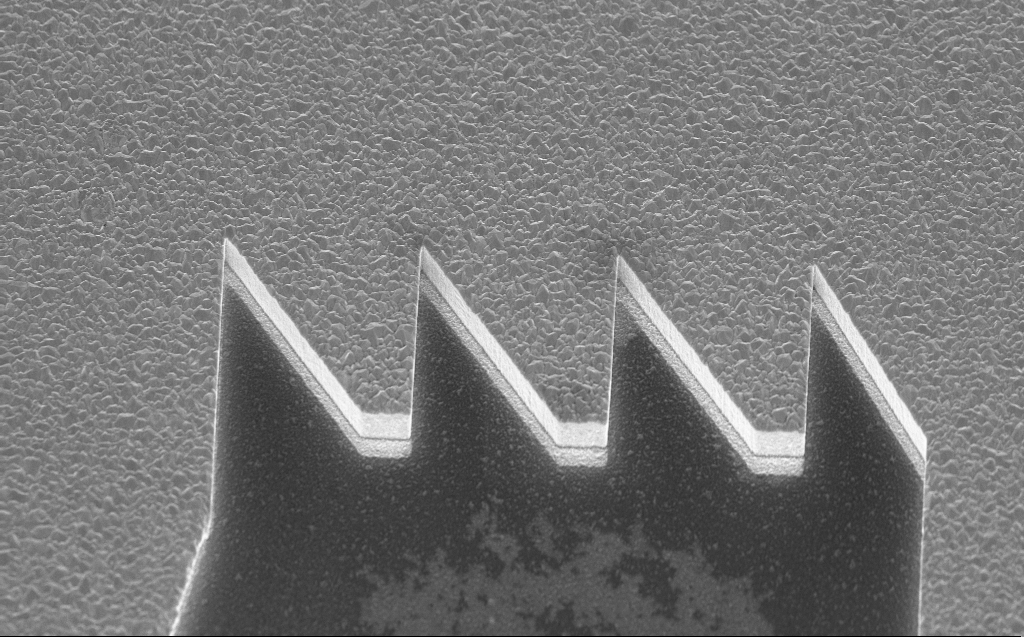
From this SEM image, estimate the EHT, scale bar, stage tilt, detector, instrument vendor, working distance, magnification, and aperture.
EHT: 10 kV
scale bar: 10000 nm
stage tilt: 45°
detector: InLens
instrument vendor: Zeiss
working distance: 5 mm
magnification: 6.3 K X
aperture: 30 µm